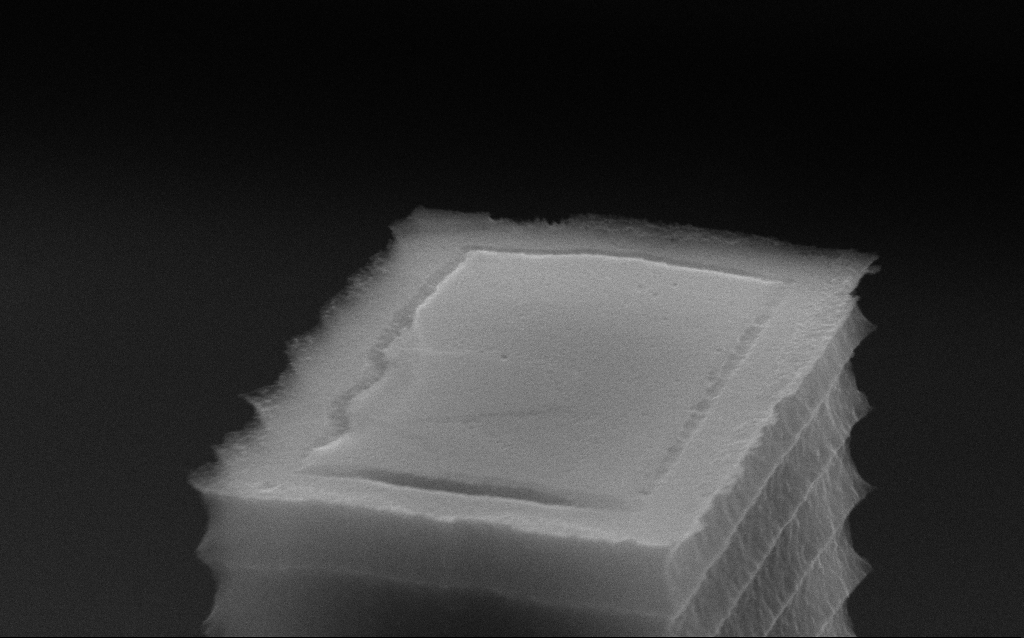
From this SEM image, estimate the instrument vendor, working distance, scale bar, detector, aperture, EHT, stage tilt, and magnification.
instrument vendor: Zeiss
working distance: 7.2 mm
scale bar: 200 nm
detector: SE2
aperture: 30 µm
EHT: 10 kV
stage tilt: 70°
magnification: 93.52 K X